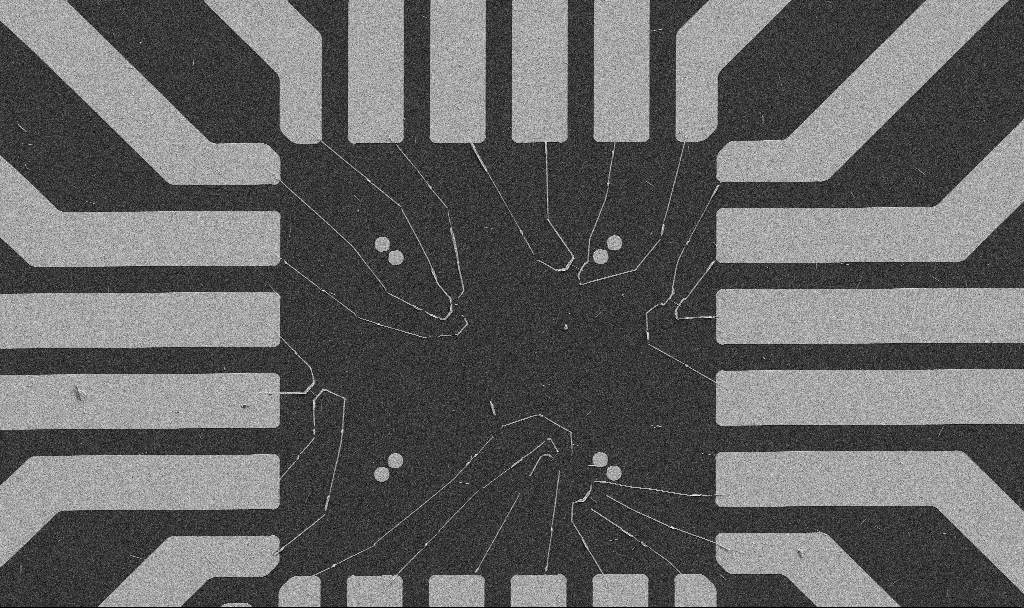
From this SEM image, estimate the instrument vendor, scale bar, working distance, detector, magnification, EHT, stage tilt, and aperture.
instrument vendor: Zeiss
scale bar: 20000 nm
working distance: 10.7 mm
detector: SE2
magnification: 1 K X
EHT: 5 kV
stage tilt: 0°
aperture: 30 µm